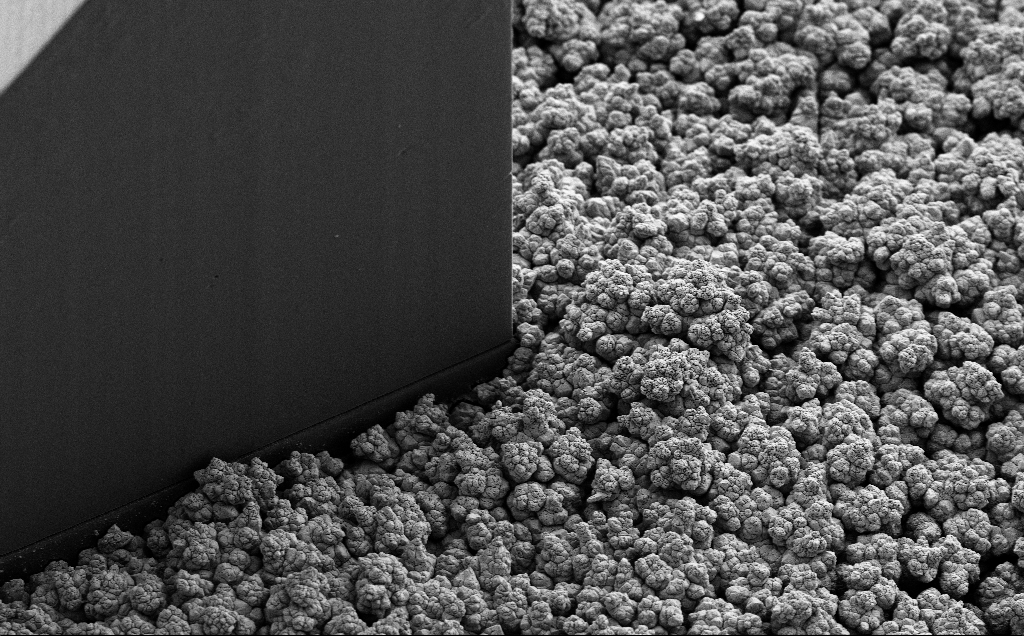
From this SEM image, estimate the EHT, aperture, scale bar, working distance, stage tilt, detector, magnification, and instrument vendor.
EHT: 5 kV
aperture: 30 µm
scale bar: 20000 nm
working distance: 9 mm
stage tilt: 35°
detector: SE2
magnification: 0.92 K X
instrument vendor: Zeiss